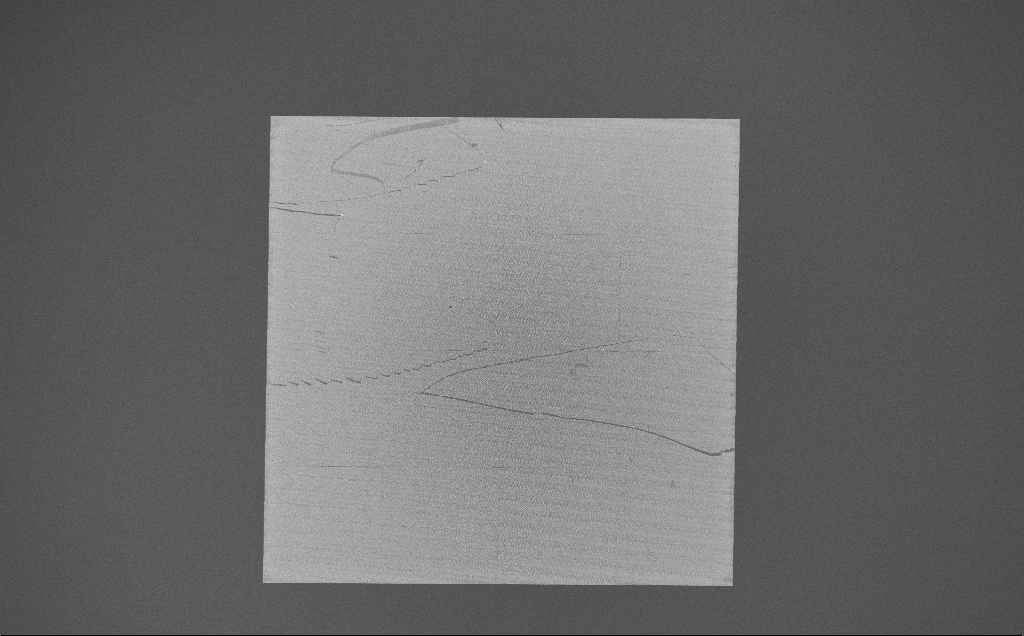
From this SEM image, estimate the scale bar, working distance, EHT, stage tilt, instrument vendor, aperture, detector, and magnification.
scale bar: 100000 nm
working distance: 7 mm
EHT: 10 kV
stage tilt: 0°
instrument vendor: Zeiss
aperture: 30 µm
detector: InLens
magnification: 0.176 K X